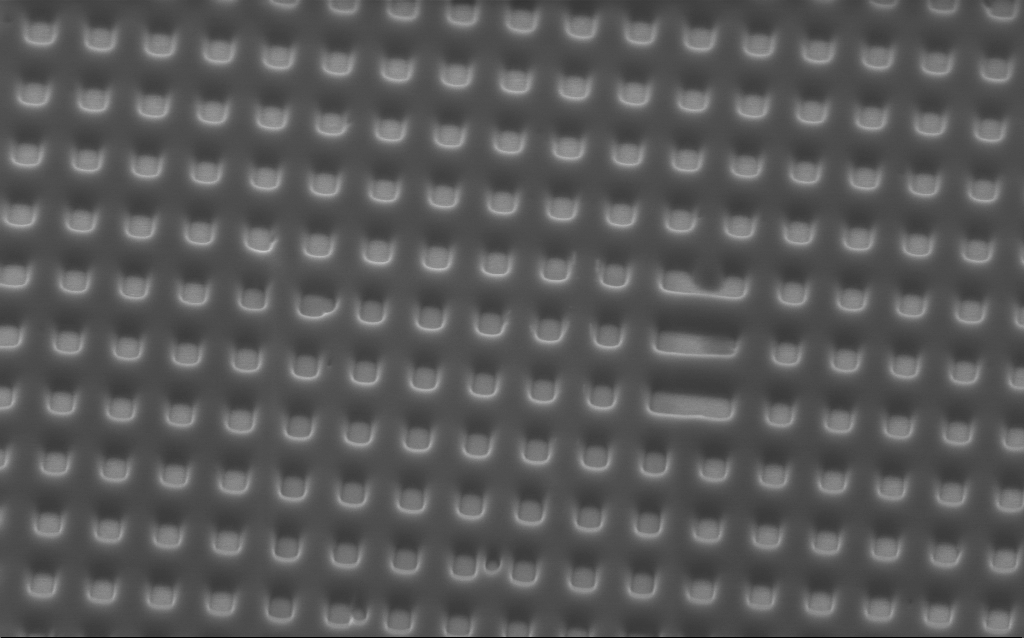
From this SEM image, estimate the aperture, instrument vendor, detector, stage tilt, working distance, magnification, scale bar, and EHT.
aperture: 30 µm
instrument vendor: Zeiss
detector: InLens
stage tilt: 45°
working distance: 2.7 mm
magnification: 44.15 K X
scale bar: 1000 nm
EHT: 2 kV